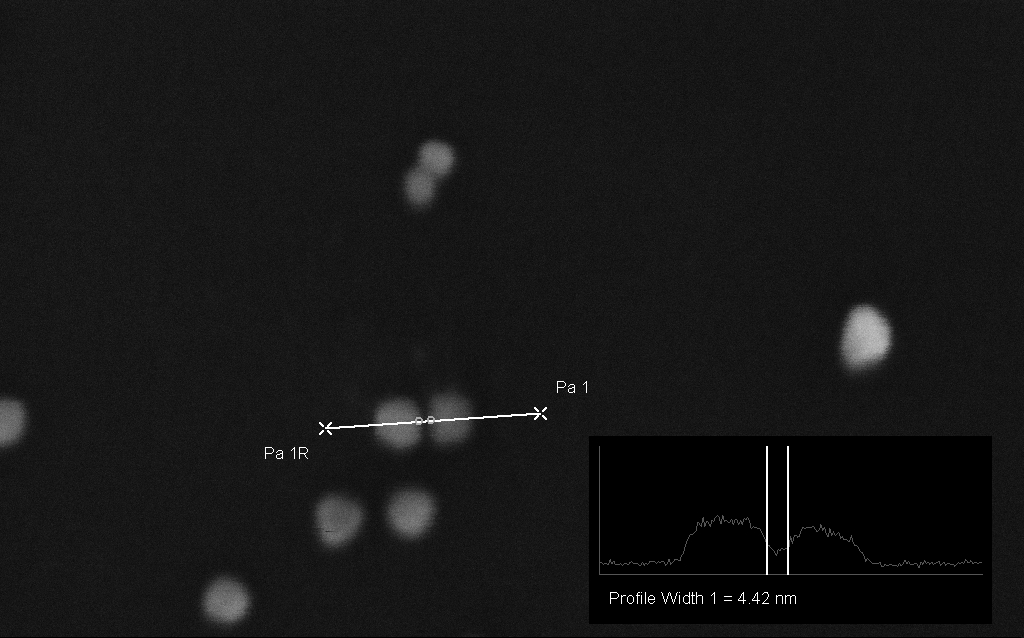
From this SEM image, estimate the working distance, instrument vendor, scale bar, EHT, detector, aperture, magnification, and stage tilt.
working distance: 1.9 mm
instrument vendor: Zeiss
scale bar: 20 nm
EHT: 10 kV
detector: InLens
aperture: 30 µm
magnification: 1000 K X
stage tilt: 0°